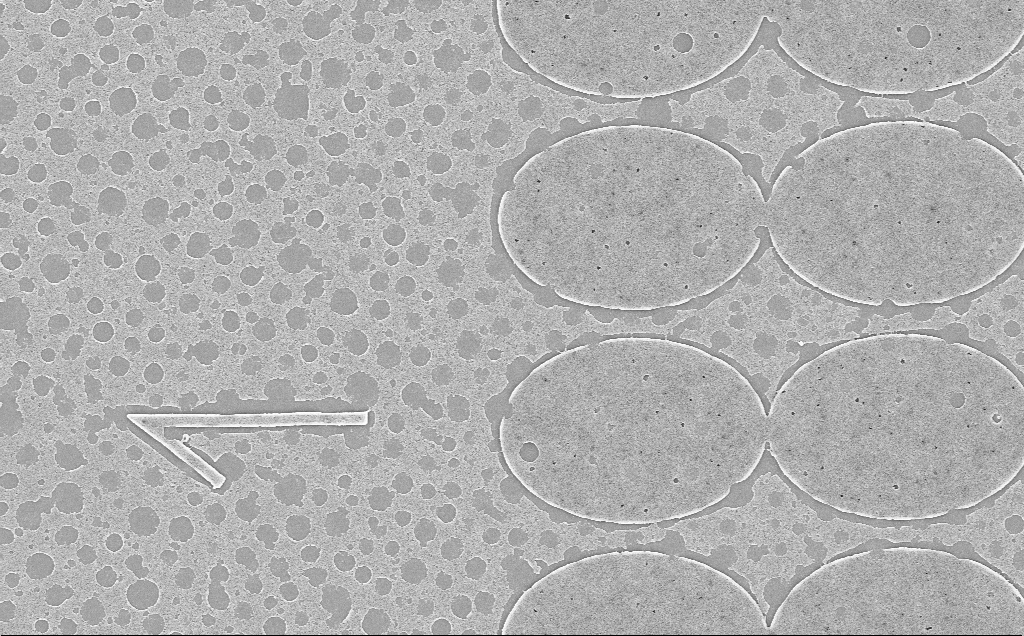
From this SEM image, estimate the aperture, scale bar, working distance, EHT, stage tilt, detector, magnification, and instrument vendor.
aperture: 30 µm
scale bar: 2000 nm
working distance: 6 mm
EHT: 5 kV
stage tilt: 0°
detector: InLens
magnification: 9.88 K X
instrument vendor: Zeiss